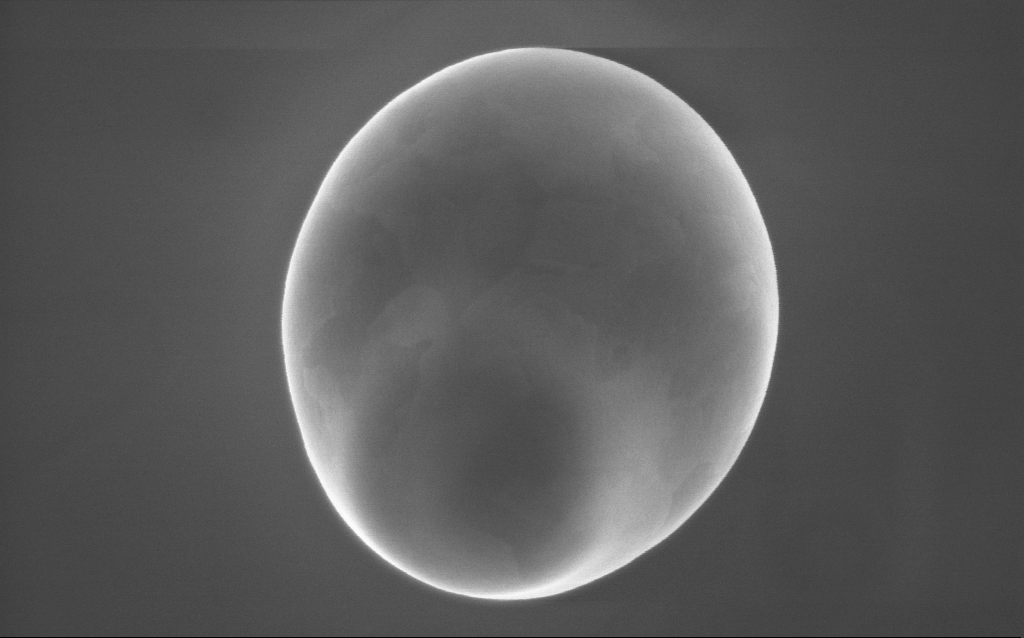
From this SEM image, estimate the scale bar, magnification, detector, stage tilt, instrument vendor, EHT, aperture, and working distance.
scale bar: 1000 nm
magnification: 71 K X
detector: InLens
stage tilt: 0°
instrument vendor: Zeiss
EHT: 10 kV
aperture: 30 µm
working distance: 2 mm